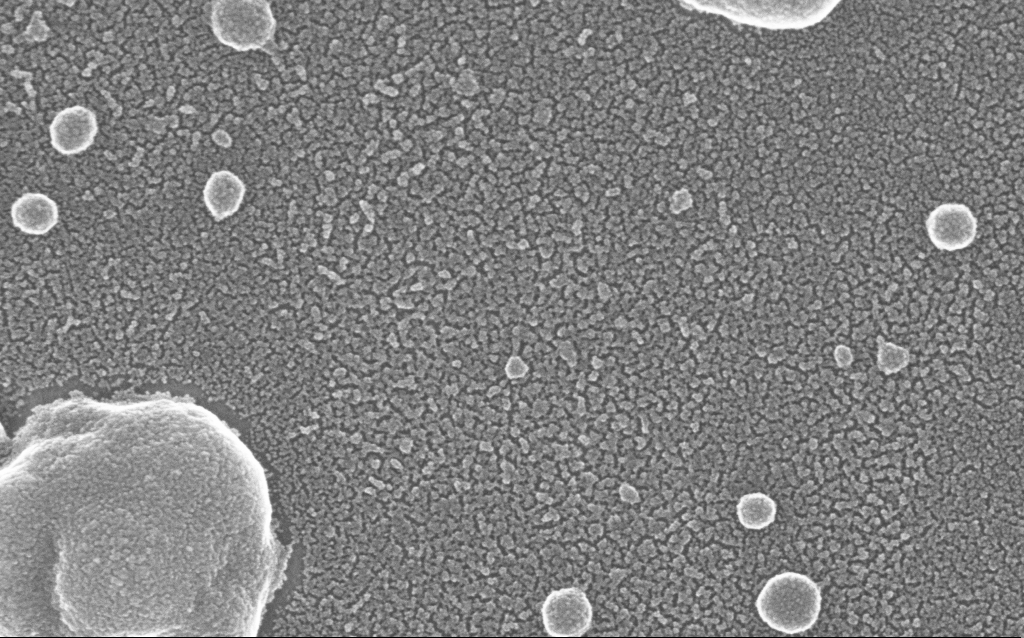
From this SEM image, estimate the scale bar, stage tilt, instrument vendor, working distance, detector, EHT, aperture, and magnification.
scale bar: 100 nm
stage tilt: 0°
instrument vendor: Zeiss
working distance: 1.5 mm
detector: InLens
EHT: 20 kV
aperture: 30 µm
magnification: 300 K X